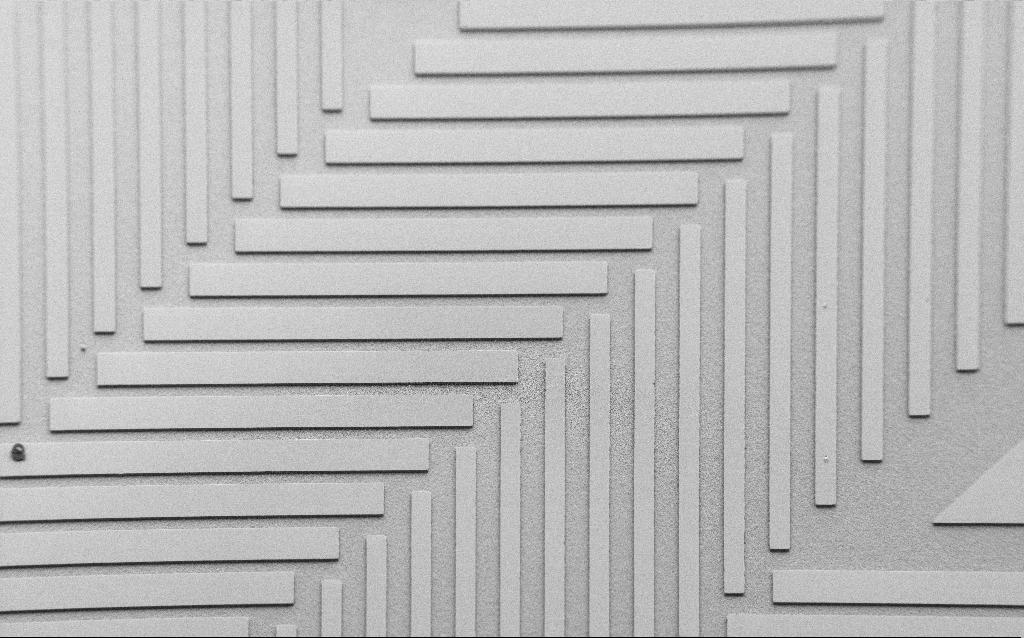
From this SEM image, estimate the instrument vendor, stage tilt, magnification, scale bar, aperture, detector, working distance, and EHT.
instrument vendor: Zeiss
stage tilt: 45°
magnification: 0.218 K X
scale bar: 100000 nm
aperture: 30 µm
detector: SE2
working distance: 7 mm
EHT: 2 kV